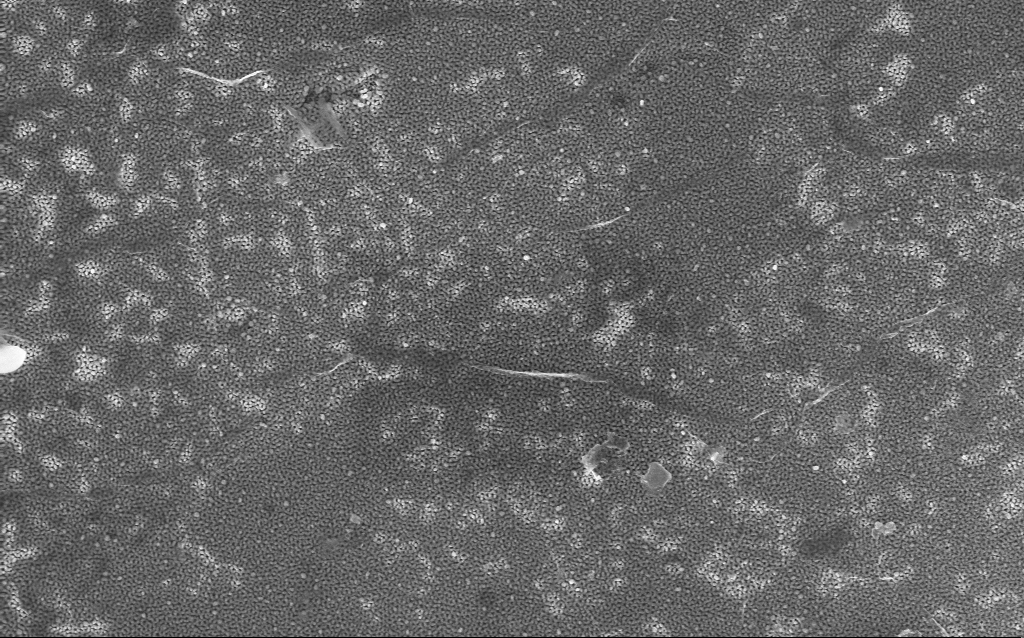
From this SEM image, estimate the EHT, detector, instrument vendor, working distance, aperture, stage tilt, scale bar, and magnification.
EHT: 5 kV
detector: InLens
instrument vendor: Zeiss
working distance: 4.1 mm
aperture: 30 µm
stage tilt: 0.1°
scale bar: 1000 nm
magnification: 48.95 K X